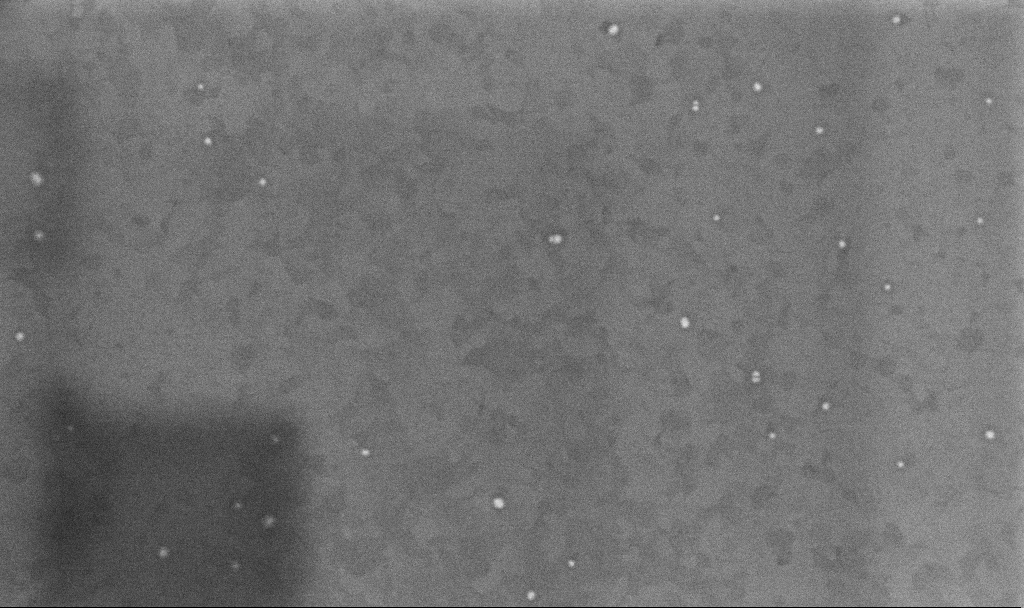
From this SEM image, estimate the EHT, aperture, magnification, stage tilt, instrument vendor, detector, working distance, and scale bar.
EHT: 10 kV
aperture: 30 µm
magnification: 100.38 K X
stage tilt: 0°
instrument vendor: Zeiss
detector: InLens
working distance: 3.3 mm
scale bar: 200 nm